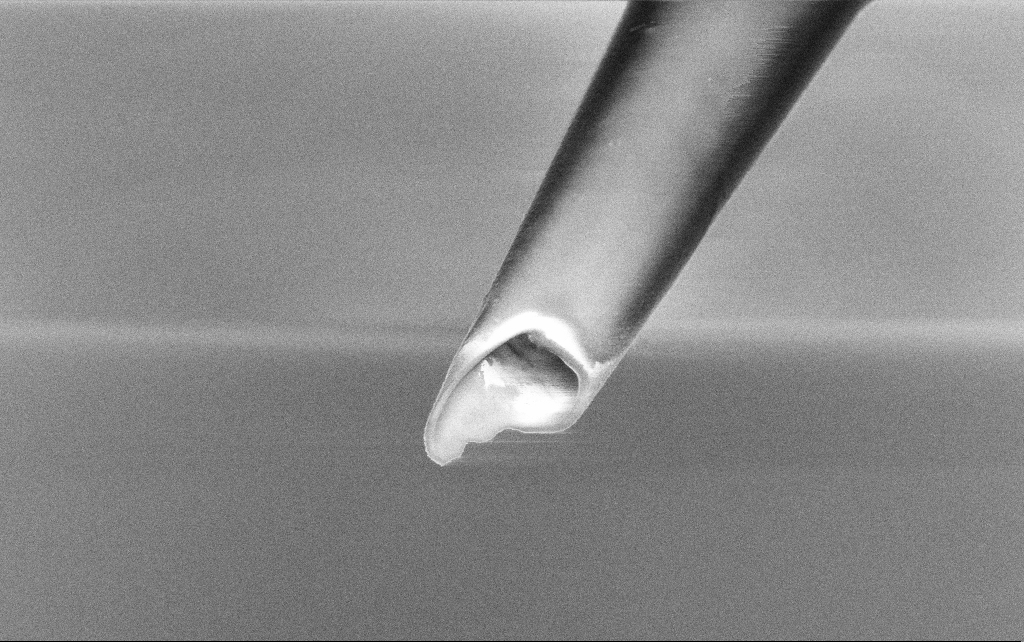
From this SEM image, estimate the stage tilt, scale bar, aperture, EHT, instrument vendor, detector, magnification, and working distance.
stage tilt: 45°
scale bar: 1000 nm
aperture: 30 µm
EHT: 3 kV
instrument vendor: Zeiss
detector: InLens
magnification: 25 K X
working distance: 7.6 mm